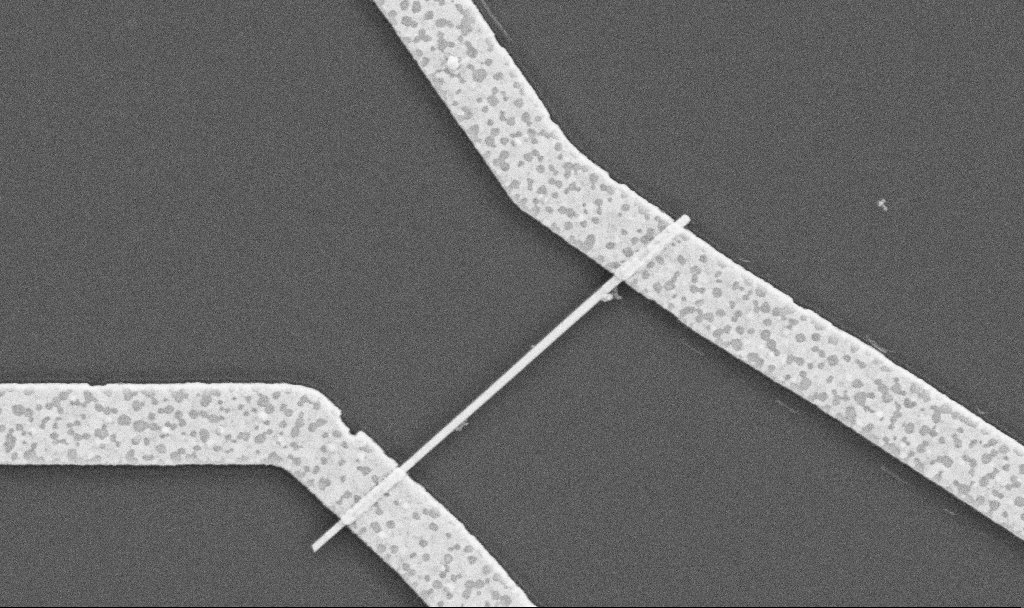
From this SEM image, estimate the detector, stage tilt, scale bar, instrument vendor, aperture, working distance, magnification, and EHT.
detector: SE2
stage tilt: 0°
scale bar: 1000 nm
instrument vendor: Zeiss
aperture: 30 µm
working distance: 10.5 mm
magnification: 30 K X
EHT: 5 kV